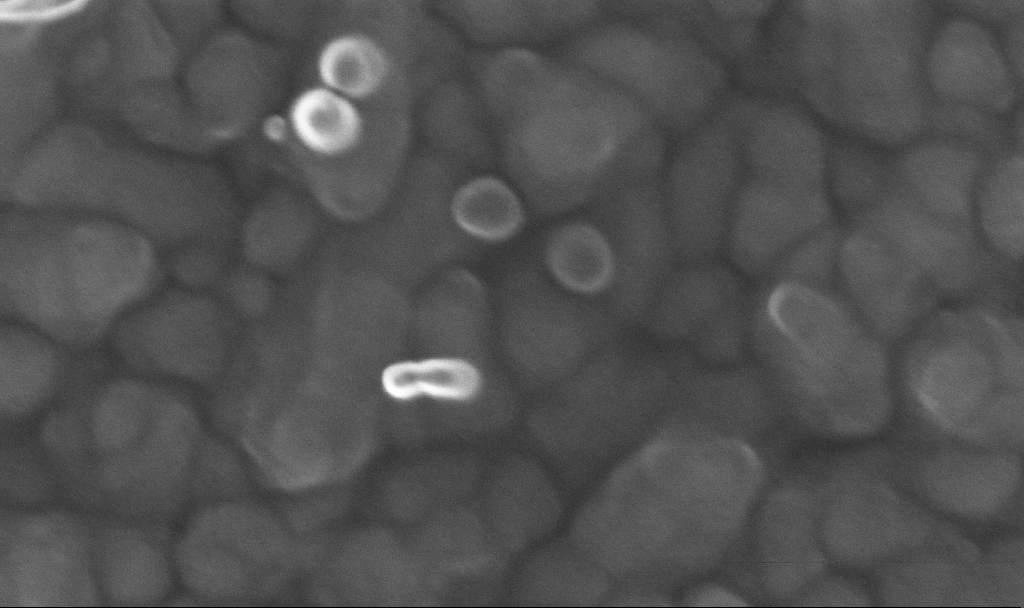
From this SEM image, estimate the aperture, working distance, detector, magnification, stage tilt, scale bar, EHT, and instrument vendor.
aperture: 30 µm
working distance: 4.8 mm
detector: InLens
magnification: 400.61 K X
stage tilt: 0°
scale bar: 100 nm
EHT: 20 kV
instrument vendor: Zeiss